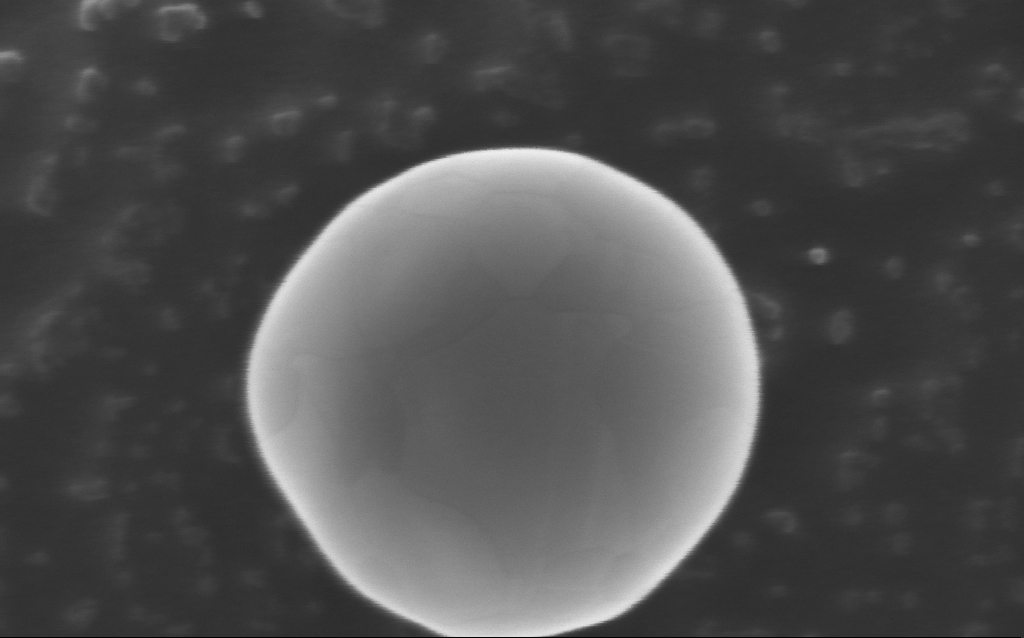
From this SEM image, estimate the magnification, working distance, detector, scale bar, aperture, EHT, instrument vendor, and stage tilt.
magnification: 404.76 K X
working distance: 5 mm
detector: InLens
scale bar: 100 nm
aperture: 30 µm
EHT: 10 kV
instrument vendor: Zeiss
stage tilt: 0°